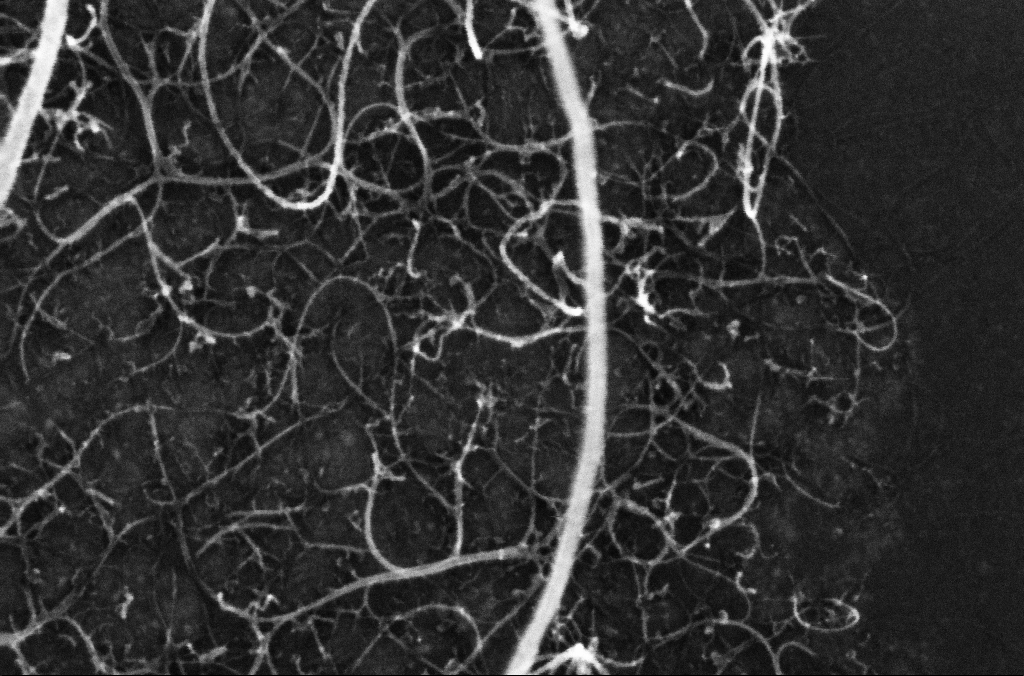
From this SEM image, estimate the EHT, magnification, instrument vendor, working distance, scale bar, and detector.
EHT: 10 kV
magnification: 307.21 K X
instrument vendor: Zeiss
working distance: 3.2 mm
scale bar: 100 nm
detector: InLens